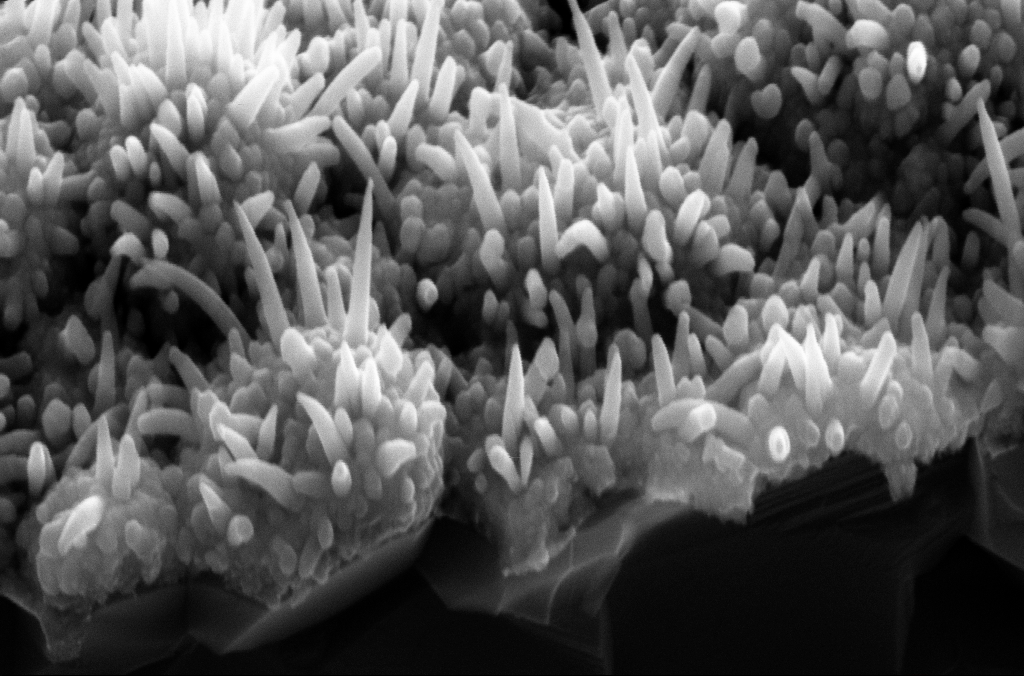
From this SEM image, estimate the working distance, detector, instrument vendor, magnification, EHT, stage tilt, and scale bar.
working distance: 8 mm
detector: SE2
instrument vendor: Zeiss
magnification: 106.23 K X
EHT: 10 kV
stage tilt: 45°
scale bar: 200 nm